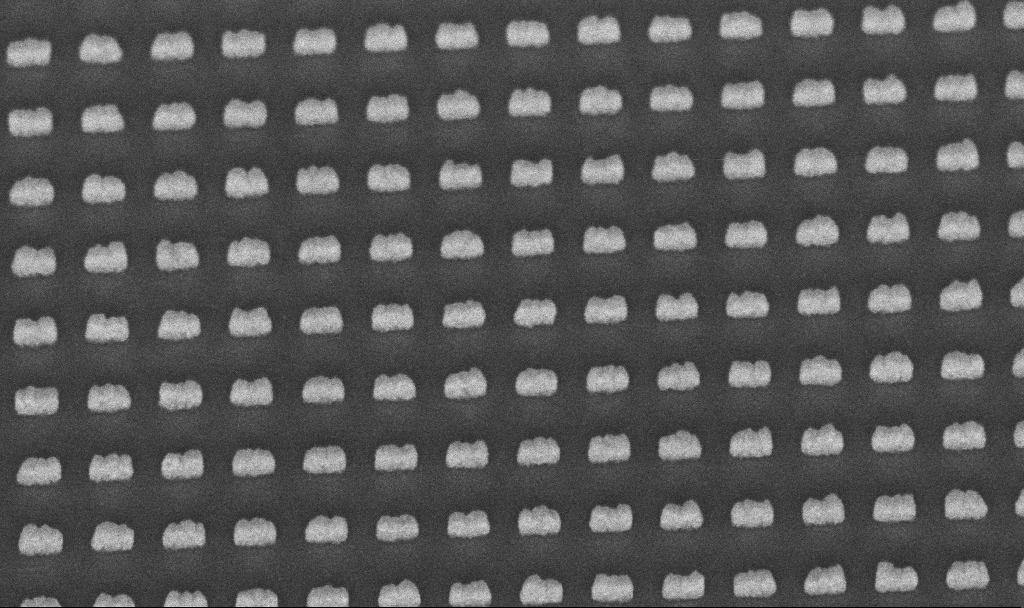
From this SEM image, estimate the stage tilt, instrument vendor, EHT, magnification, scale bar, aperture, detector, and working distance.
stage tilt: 45°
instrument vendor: Zeiss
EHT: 5 kV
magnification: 113.27 K X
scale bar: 200 nm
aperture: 30 µm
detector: InLens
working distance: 6.7 mm